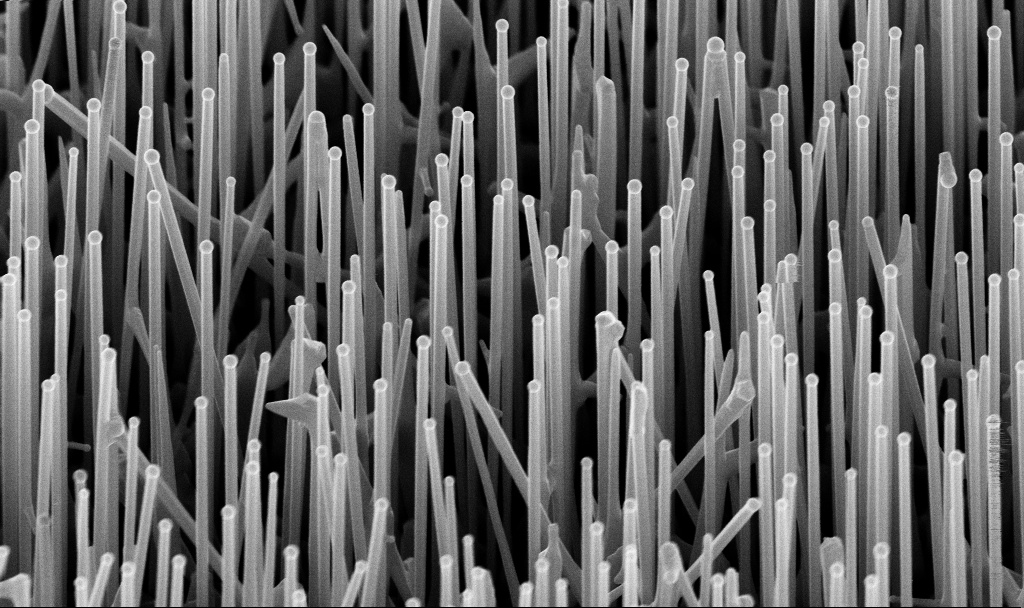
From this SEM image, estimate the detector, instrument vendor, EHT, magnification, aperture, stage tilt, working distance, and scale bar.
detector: InLens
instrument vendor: Zeiss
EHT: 10 kV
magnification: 38.91 K X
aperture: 30 µm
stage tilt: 45°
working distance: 5.5 mm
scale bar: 1000 nm